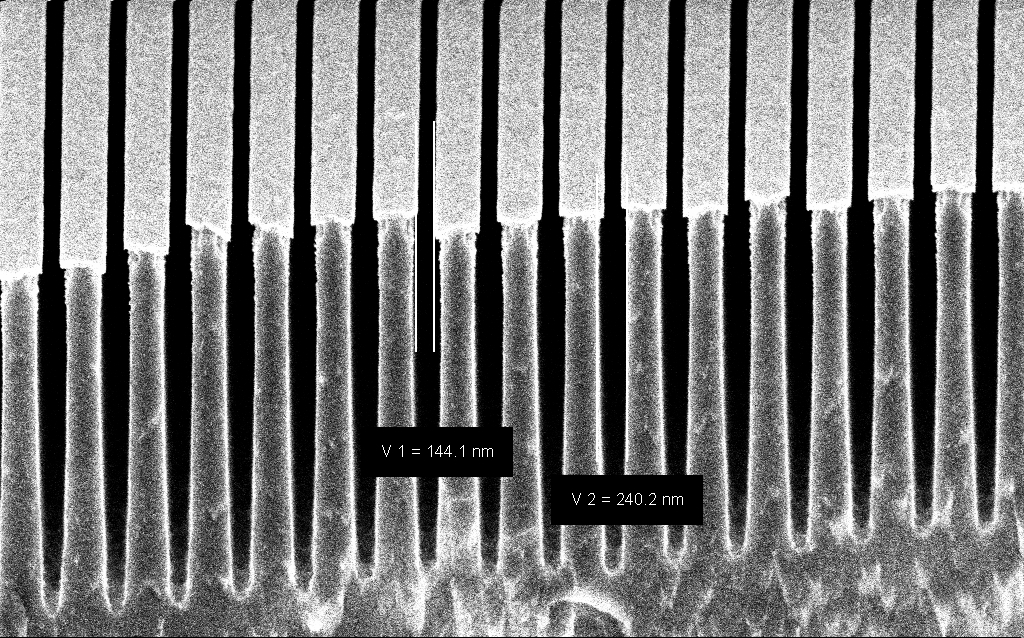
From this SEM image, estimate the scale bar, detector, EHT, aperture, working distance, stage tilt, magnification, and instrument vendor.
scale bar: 1000 nm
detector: InLens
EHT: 3 kV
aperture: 30 µm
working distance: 6 mm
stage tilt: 45°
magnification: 45.85 K X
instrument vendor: Zeiss